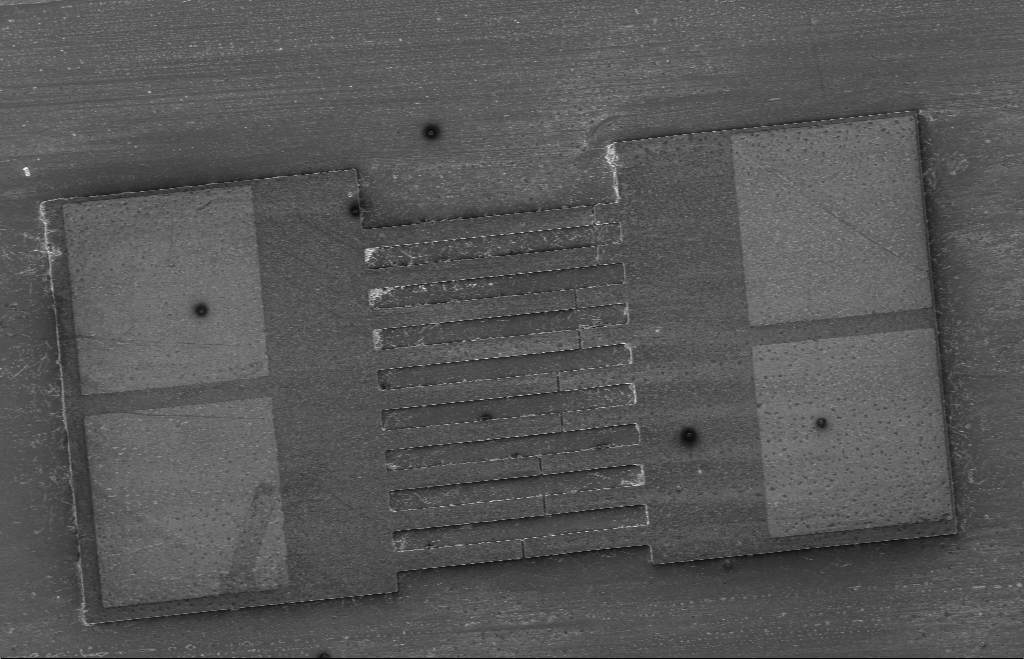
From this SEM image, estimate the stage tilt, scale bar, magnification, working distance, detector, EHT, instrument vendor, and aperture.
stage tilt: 0.1°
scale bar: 100000 nm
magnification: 0.376 K X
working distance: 11 mm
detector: InLens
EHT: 5 kV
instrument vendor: Zeiss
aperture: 30 µm